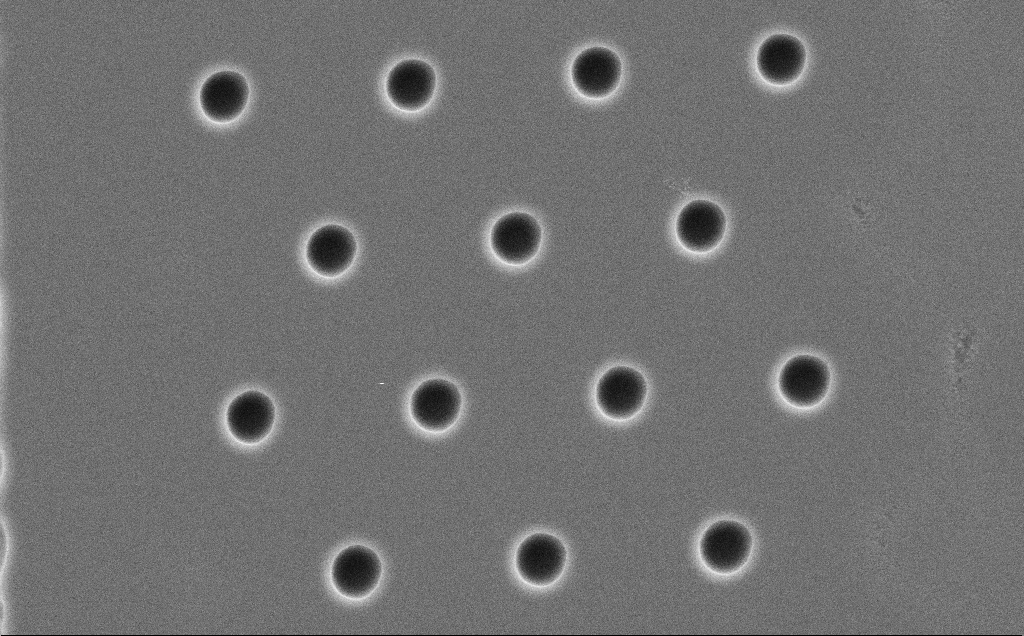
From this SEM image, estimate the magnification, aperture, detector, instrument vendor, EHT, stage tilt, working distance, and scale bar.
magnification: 10.62 K X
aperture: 30 µm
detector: SE2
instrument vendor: Zeiss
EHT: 5 kV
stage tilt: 0°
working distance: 13 mm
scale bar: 2000 nm